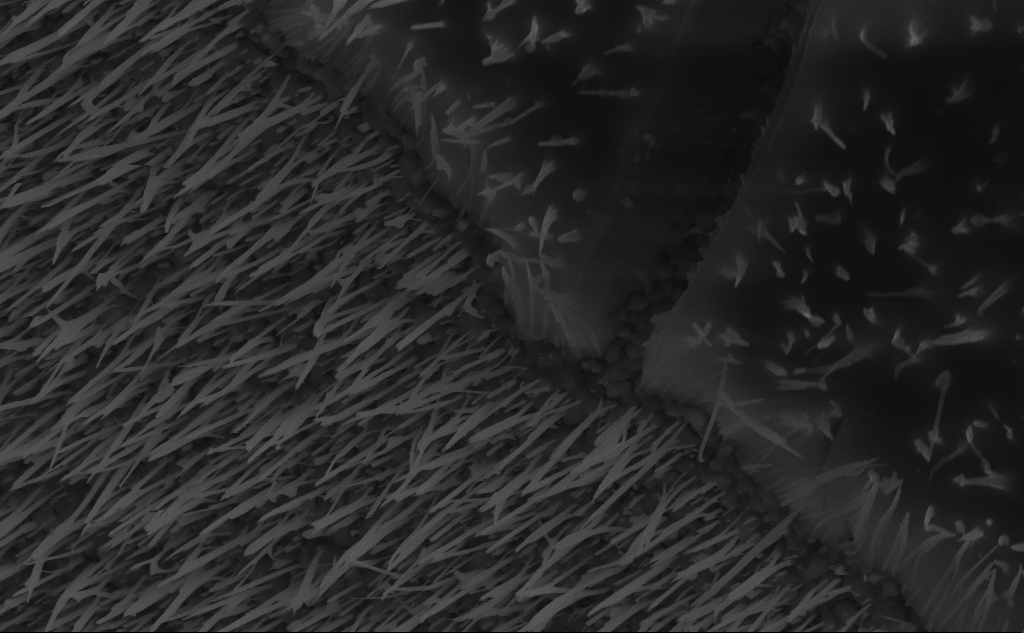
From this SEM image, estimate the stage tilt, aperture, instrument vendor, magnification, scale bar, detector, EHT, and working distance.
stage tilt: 45°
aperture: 30 µm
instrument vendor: Zeiss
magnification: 35.6 K X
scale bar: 2000 nm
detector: InLens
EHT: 10 kV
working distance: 6 mm